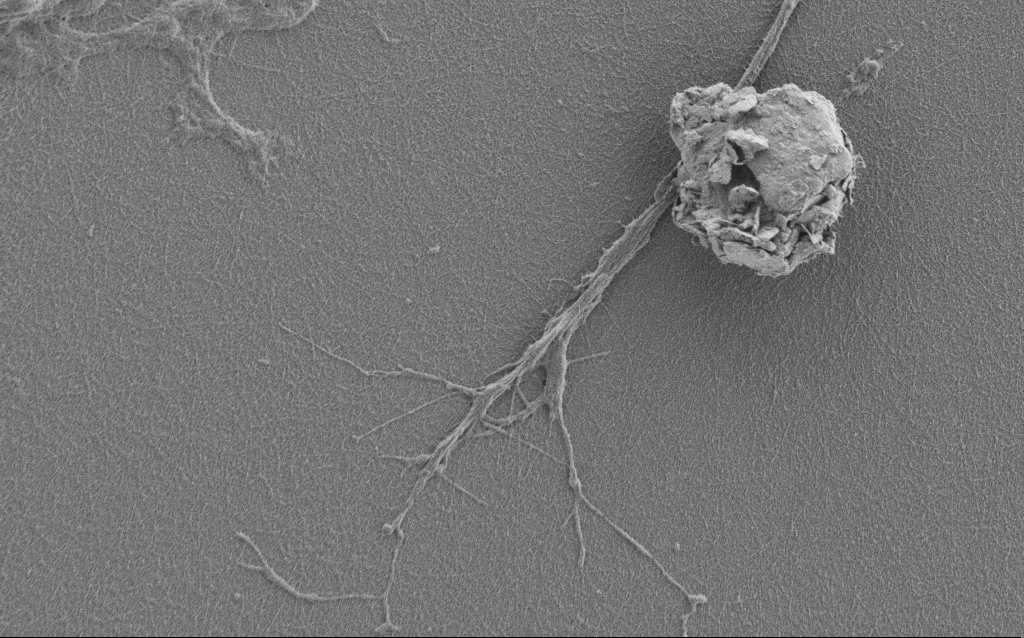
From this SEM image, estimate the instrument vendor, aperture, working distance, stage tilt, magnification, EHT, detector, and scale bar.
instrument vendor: Zeiss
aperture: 30 µm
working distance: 6 mm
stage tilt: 0°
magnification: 7.5 K X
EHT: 1 kV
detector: SE2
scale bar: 2000 nm